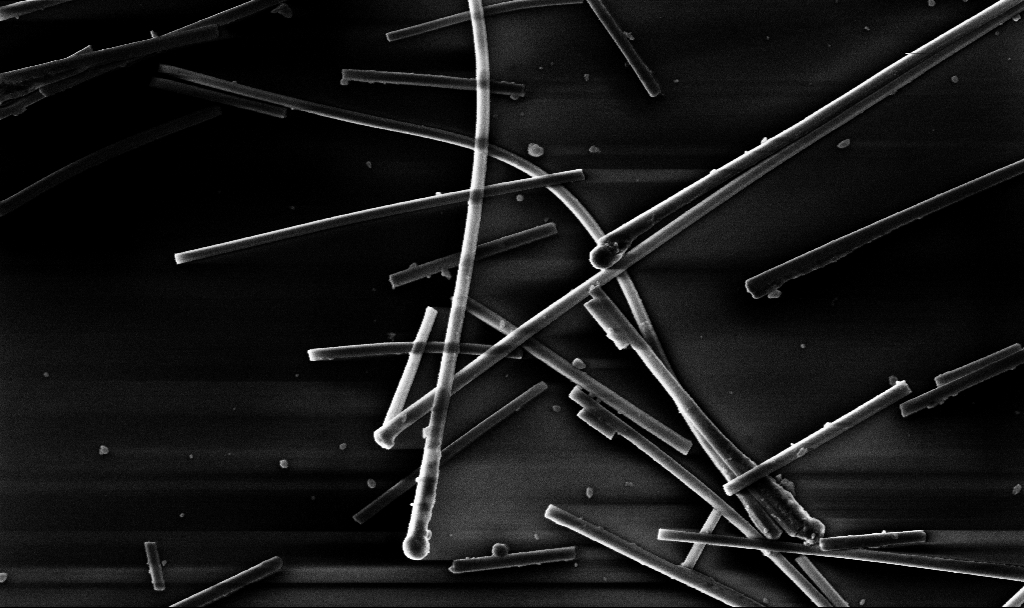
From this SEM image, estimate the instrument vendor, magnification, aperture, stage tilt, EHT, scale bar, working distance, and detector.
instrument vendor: Zeiss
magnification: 43.77 K X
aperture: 30 µm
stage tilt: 0°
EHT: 5 kV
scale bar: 1000 nm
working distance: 10.7 mm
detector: InLens